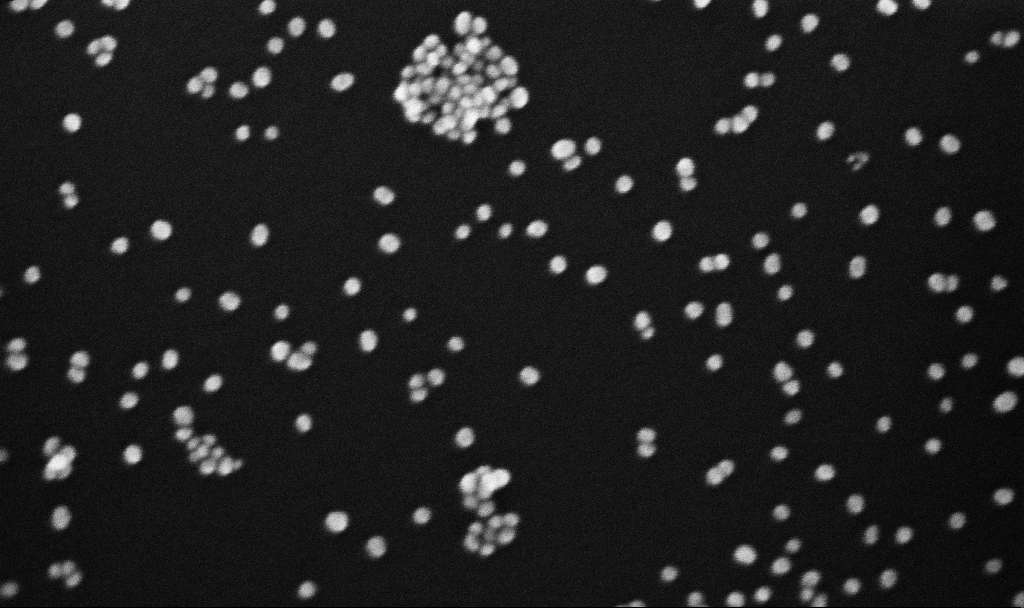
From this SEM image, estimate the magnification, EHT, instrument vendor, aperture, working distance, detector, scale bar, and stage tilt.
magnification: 300 K X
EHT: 10 kV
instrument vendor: Zeiss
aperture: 30 µm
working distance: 5.4 mm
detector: InLens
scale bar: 200 nm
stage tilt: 0°